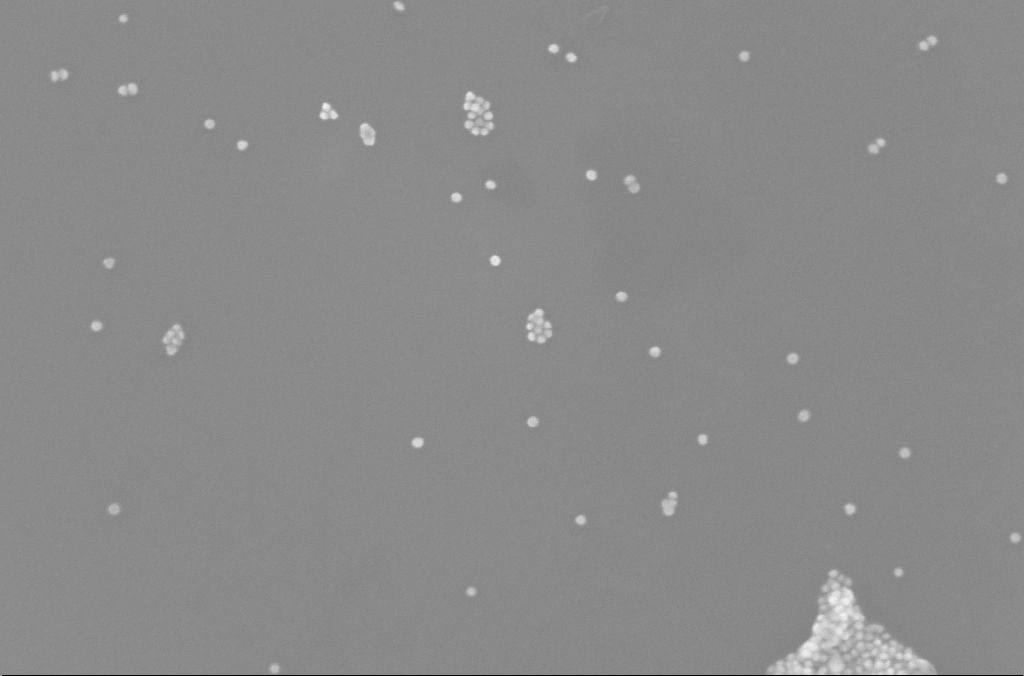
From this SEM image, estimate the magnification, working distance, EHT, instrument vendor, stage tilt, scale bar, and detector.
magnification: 181.64 K X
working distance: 2.5 mm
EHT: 10 kV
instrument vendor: Zeiss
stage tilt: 0°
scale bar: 200 nm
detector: InLens